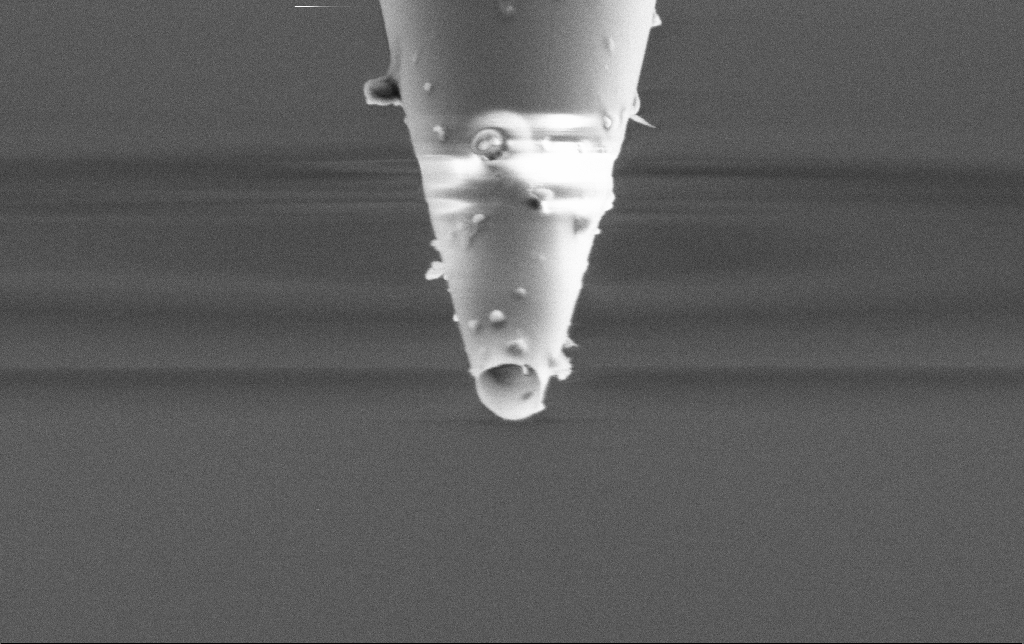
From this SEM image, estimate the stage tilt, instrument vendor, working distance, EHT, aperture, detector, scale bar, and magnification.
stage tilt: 45°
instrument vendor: Zeiss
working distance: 7.4 mm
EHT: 2 kV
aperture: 30 µm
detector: SE2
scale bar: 1000 nm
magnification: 50 K X